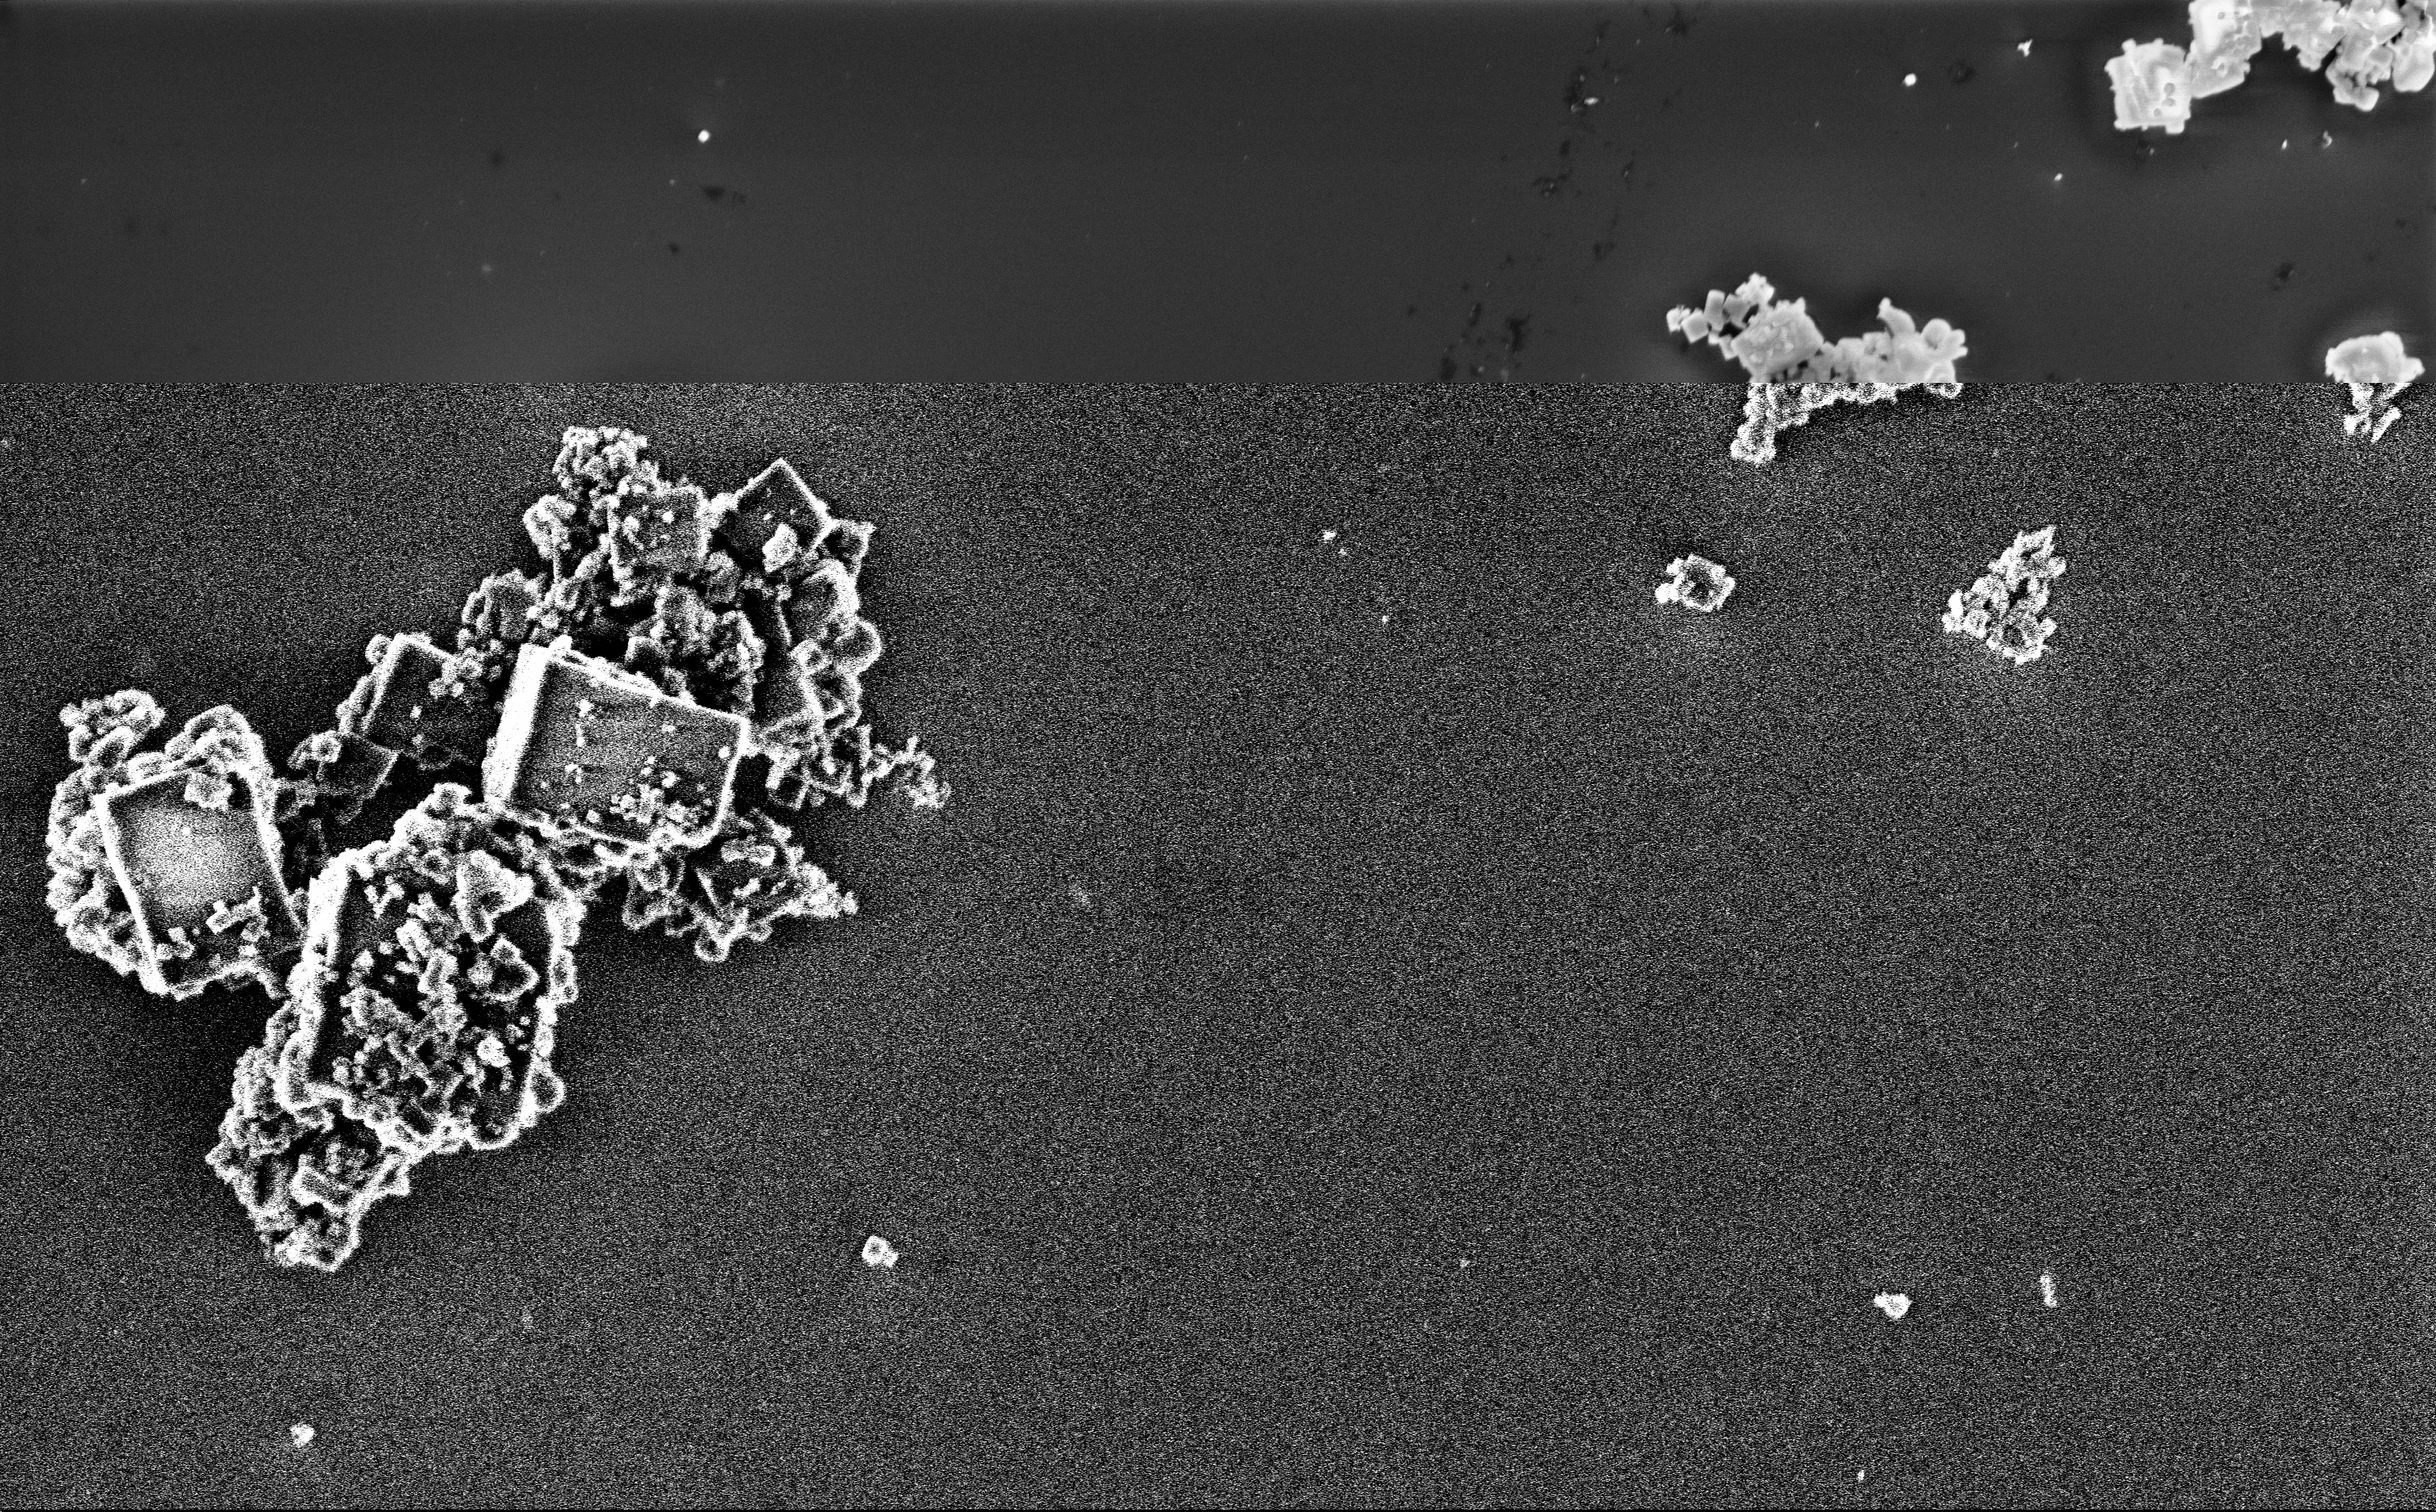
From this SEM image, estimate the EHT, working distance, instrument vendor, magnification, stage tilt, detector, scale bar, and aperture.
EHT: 3 kV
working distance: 3 mm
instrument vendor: Zeiss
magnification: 13.53 K X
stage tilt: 0°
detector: InLens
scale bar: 2000 nm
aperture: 30 µm